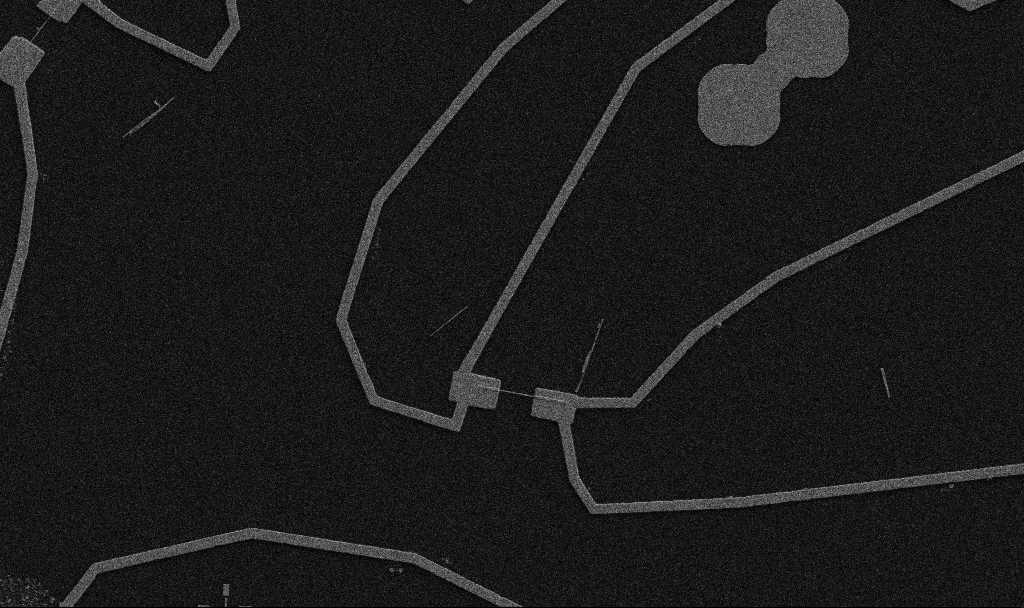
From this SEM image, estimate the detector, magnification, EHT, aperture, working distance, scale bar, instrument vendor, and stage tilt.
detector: SE2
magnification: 5 K X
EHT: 5 kV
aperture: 30 µm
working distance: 10.7 mm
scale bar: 10000 nm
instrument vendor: Zeiss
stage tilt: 0°